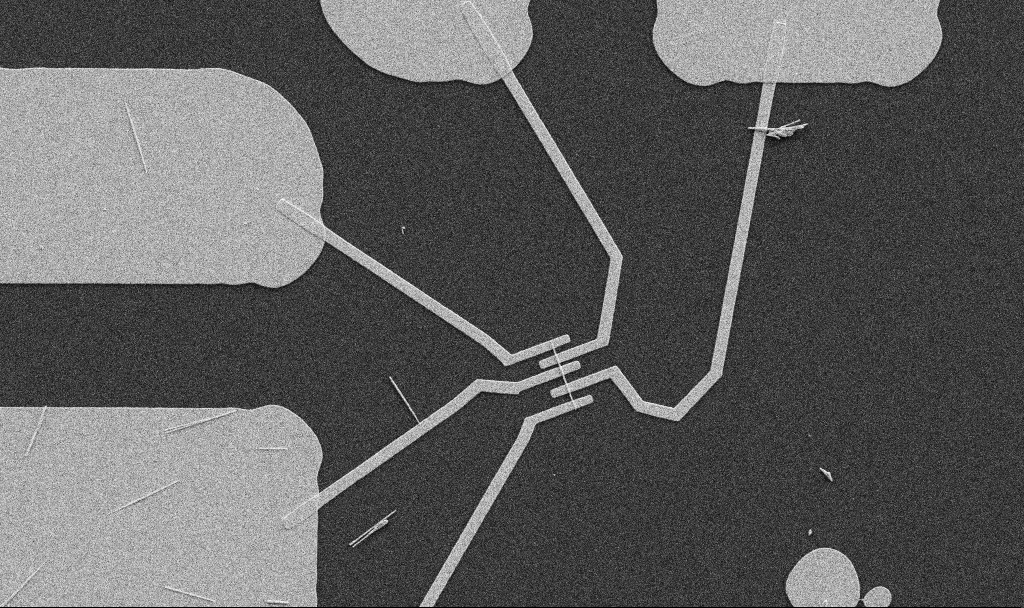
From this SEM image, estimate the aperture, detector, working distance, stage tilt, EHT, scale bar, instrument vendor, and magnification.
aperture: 30 µm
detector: SE2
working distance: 10.7 mm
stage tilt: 0°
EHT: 5 kV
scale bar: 10000 nm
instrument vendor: Zeiss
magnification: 5 K X